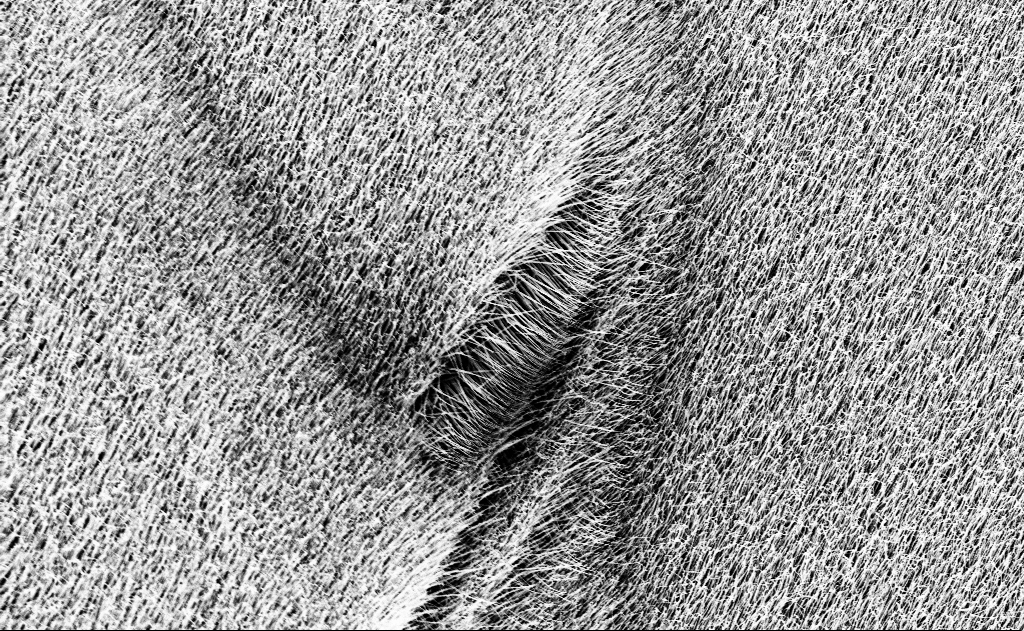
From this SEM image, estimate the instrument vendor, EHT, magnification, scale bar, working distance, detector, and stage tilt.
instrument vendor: Zeiss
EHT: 10 kV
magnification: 5 K X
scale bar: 10000 nm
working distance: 13 mm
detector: InLens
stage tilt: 0°